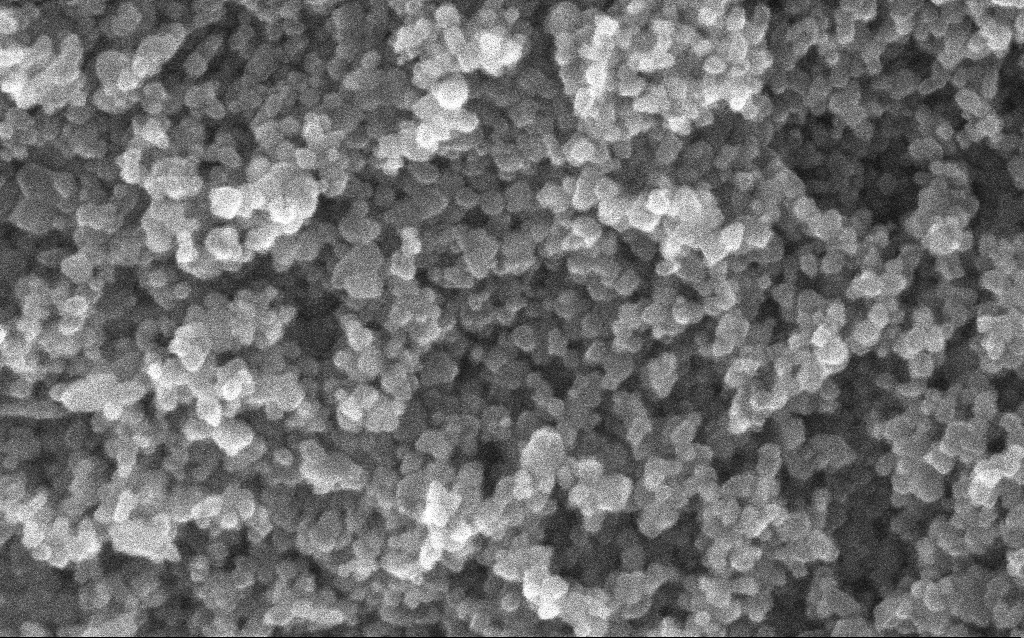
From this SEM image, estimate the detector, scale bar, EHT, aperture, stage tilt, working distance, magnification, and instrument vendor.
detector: InLens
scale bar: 100 nm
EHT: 10 kV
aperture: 30 µm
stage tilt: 0°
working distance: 2.6 mm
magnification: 348.1 K X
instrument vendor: Zeiss